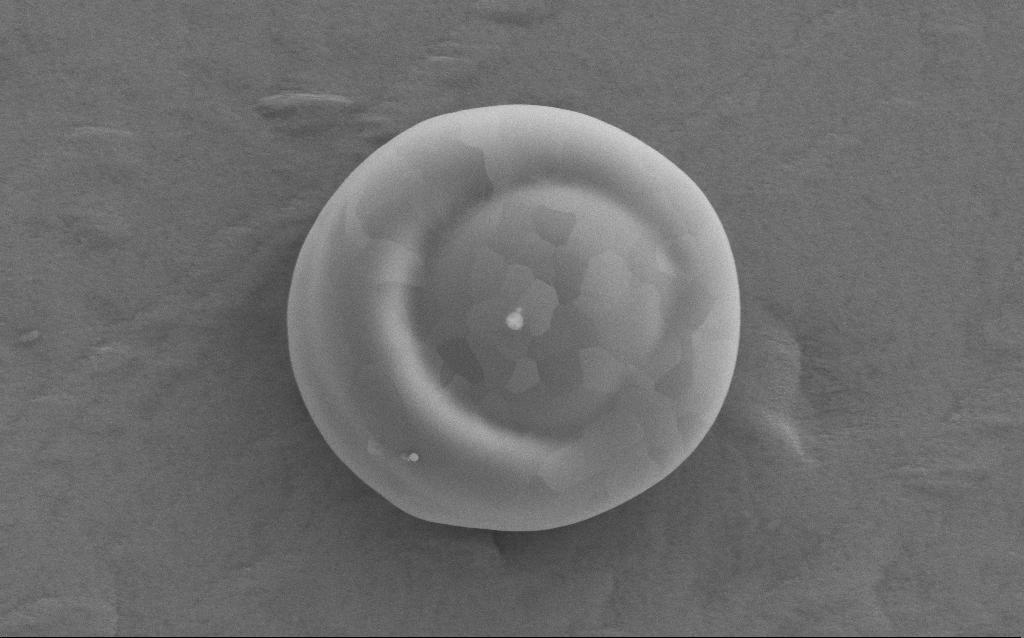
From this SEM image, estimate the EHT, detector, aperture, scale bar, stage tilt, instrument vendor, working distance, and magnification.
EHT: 5 kV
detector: SE2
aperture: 30 µm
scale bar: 1000 nm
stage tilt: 0°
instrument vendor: Zeiss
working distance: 4 mm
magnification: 36.96 K X